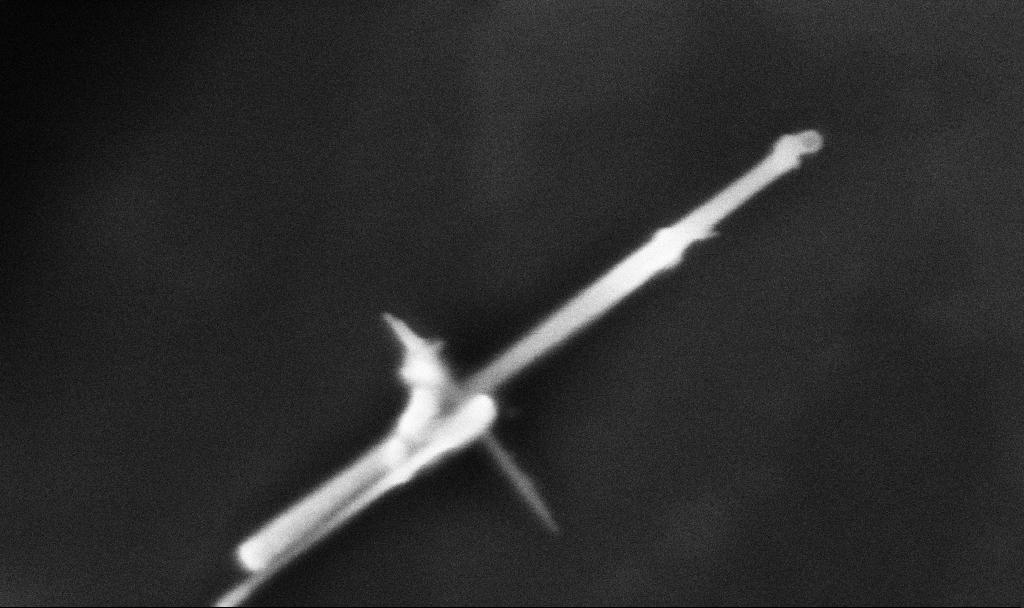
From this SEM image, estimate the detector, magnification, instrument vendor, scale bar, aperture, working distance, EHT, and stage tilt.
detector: InLens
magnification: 180.66 K X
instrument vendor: Zeiss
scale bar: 200 nm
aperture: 30 µm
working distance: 3.3 mm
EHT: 3 kV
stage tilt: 0°